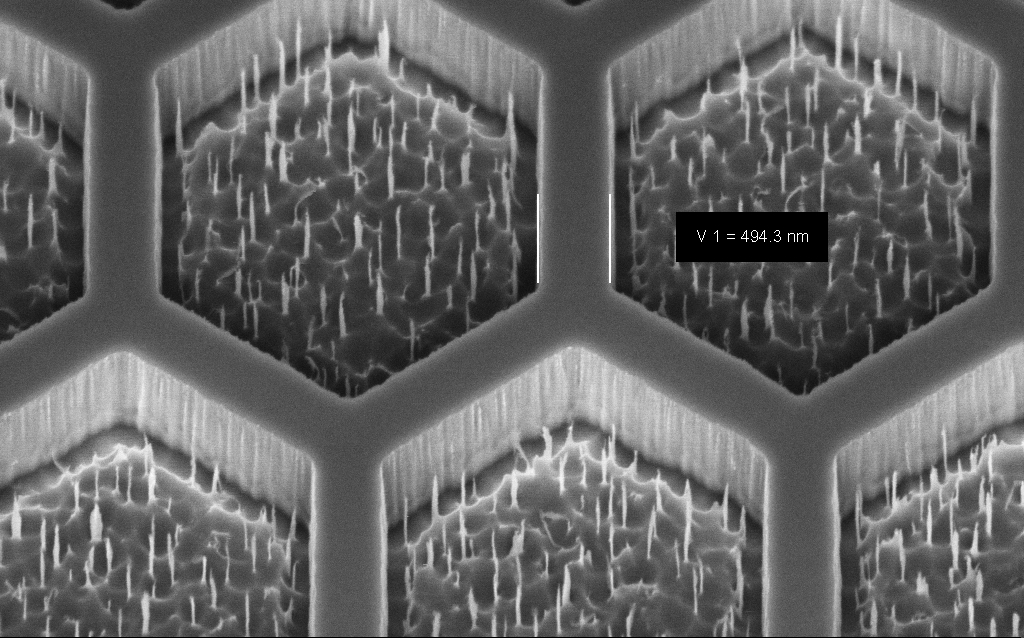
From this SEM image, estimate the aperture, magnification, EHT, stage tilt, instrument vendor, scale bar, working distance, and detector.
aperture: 30 µm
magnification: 53.48 K X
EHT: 3 kV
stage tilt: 45°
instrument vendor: Zeiss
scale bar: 1000 nm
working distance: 8 mm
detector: InLens